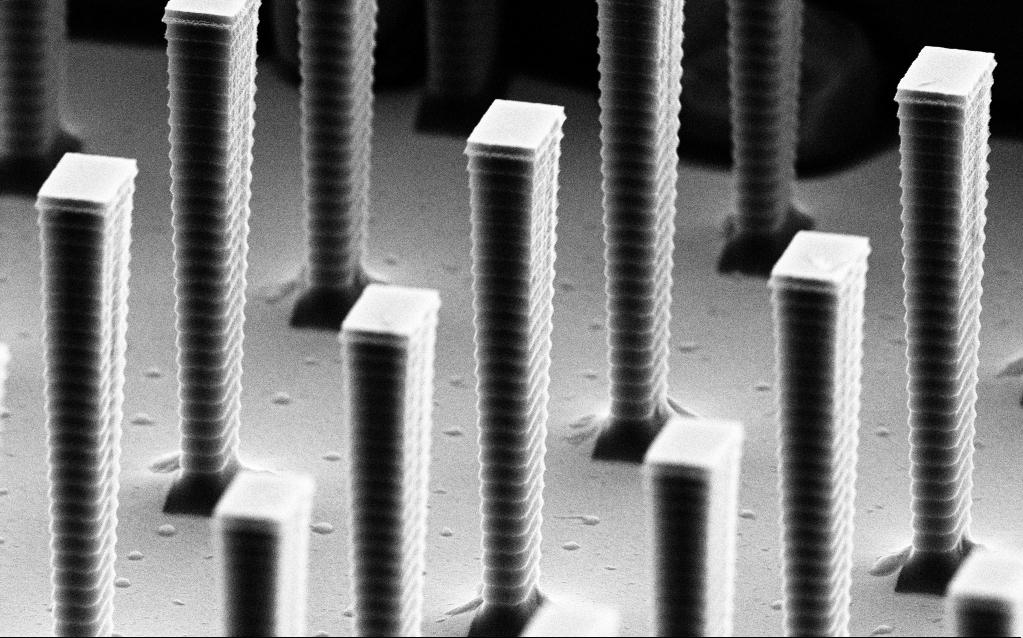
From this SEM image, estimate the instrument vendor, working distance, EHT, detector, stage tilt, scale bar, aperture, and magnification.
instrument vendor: Zeiss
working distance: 8.5 mm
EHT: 8 kV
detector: SE2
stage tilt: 70°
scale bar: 1000 nm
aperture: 30 µm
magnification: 15.36 K X